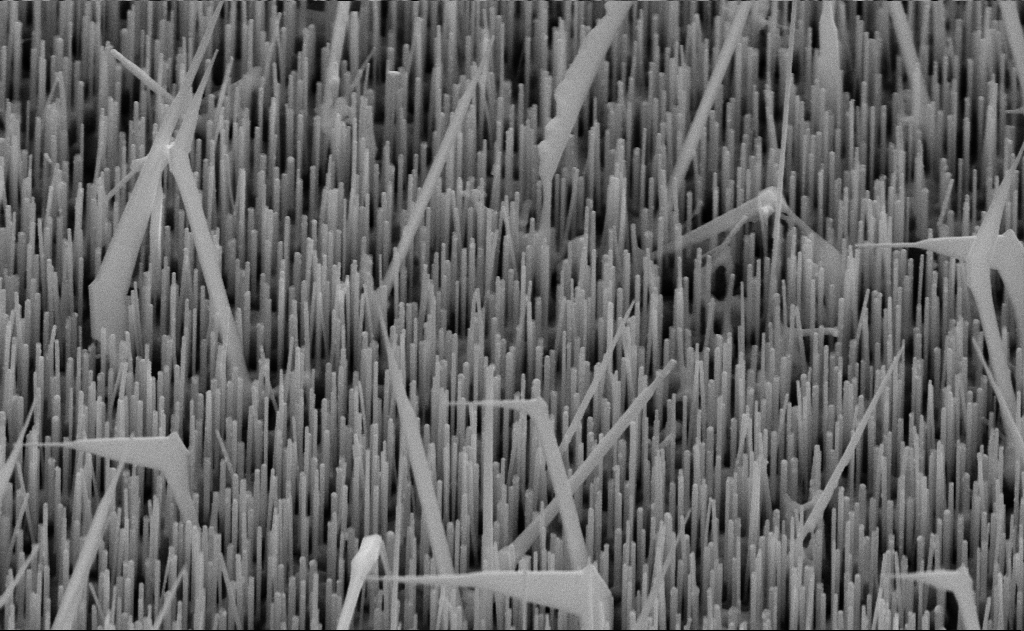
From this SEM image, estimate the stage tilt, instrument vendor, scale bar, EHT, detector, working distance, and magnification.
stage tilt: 45°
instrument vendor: Zeiss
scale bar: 1000 nm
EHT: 10 kV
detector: SE2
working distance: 11 mm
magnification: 40 K X